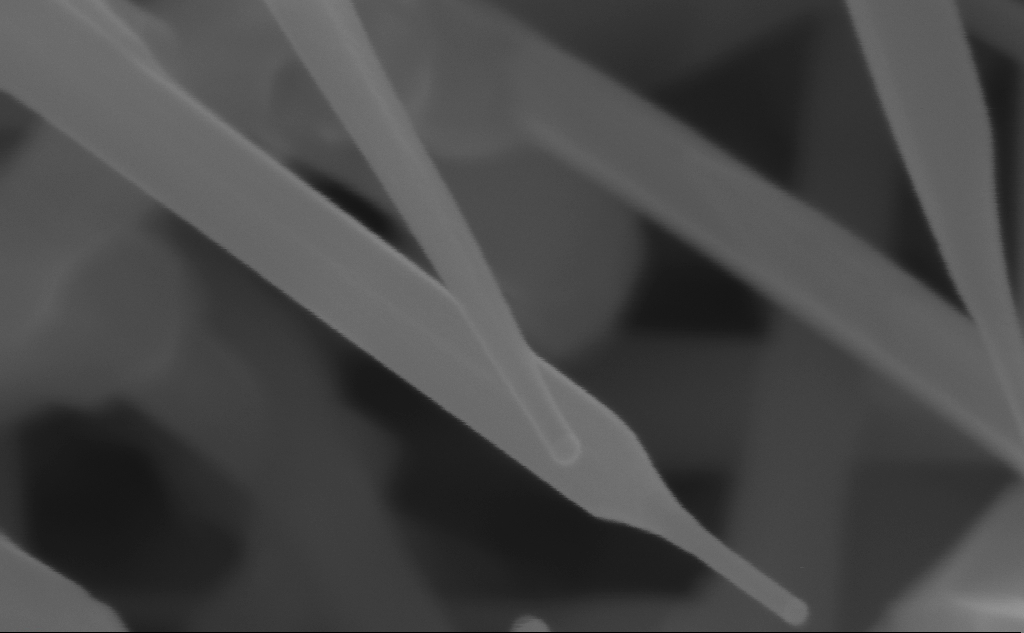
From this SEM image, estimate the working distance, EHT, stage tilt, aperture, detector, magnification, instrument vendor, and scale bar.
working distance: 7 mm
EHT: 10 kV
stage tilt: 0°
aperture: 30 µm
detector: InLens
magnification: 416.18 K X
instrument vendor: Zeiss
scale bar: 100 nm